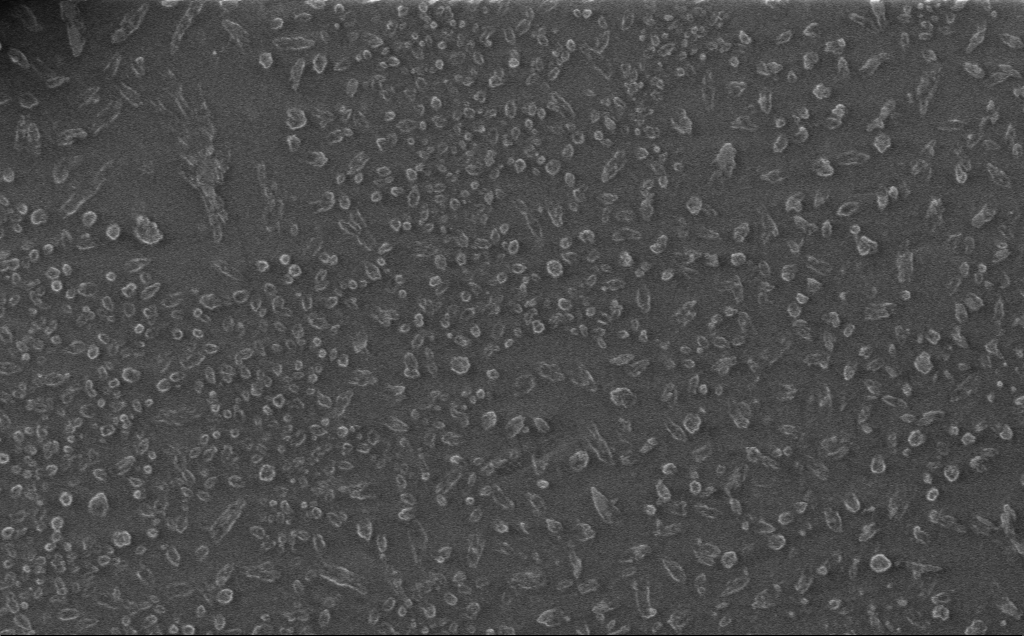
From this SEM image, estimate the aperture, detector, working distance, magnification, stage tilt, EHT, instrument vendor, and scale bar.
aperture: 30 µm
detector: InLens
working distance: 3 mm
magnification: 7.19 K X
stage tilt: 0°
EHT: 1 kV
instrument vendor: Zeiss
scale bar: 10000 nm